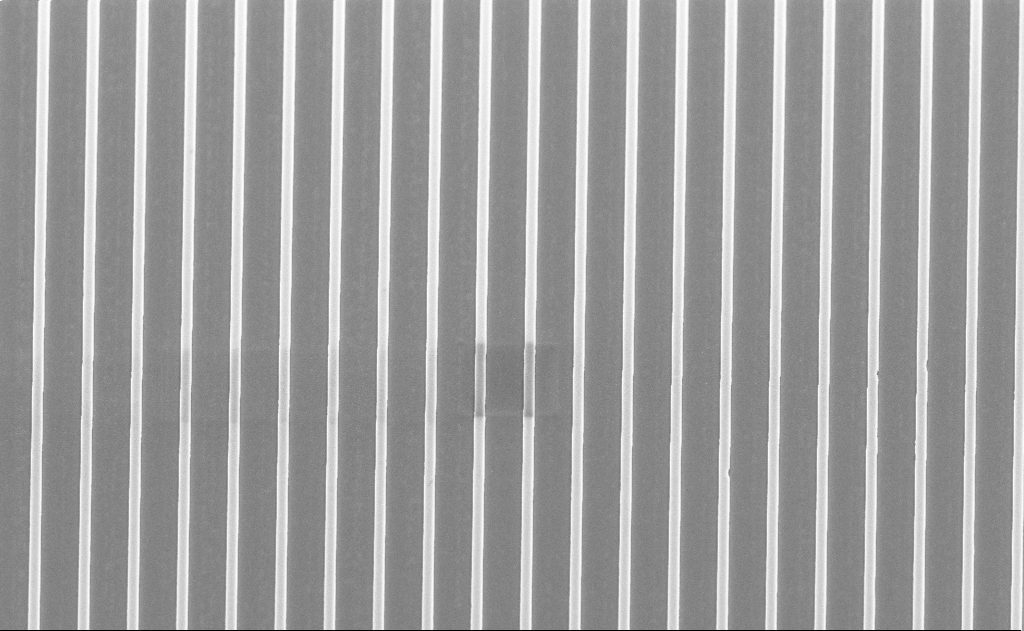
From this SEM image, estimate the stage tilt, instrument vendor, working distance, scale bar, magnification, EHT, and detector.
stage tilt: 55°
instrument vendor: Zeiss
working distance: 13 mm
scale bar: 10000 nm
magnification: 4.5 K X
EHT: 5 kV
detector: SE2